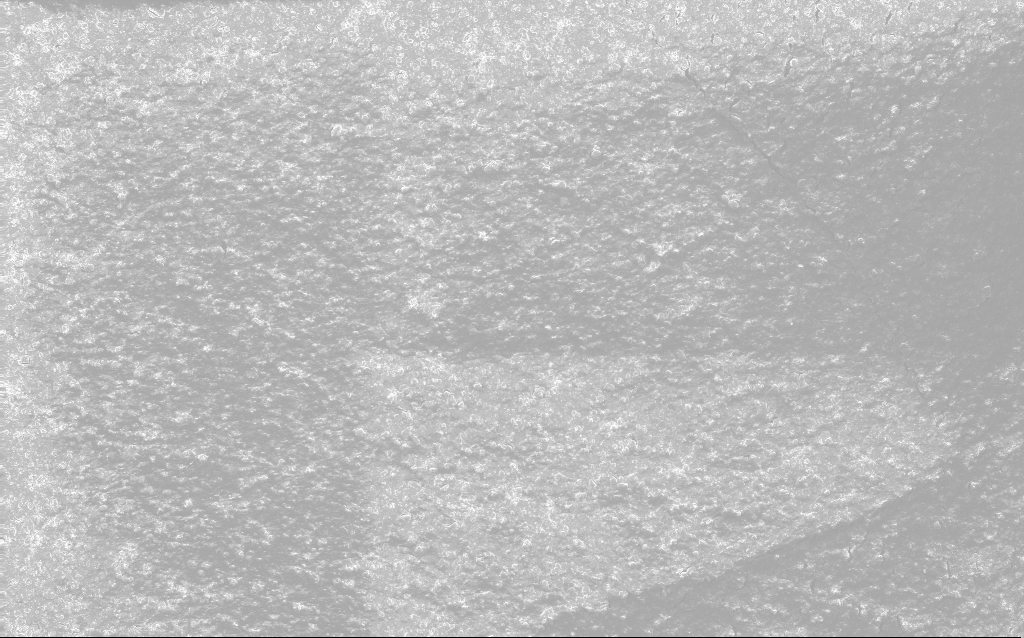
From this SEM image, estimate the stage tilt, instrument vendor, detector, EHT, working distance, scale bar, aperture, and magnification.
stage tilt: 0°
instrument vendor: Zeiss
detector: InLens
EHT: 10 kV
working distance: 2.7 mm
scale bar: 100000 nm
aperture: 30 µm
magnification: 0.235 K X